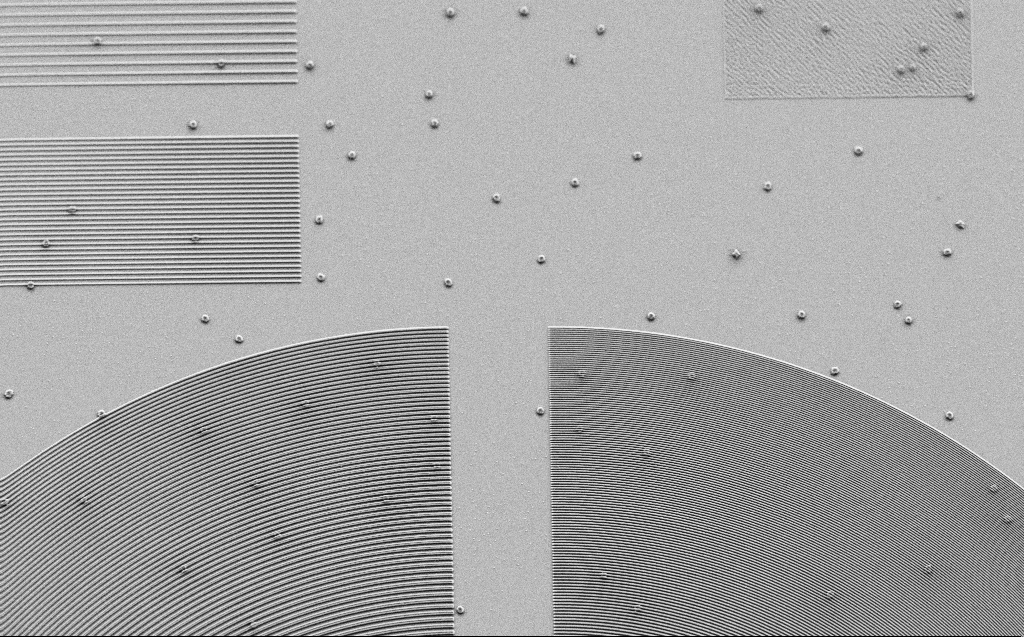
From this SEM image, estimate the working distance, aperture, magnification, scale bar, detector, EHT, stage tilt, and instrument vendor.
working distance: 6 mm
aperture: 30 µm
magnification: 3.71 K X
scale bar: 10000 nm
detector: SE2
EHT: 3 kV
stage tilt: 30°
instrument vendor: Zeiss